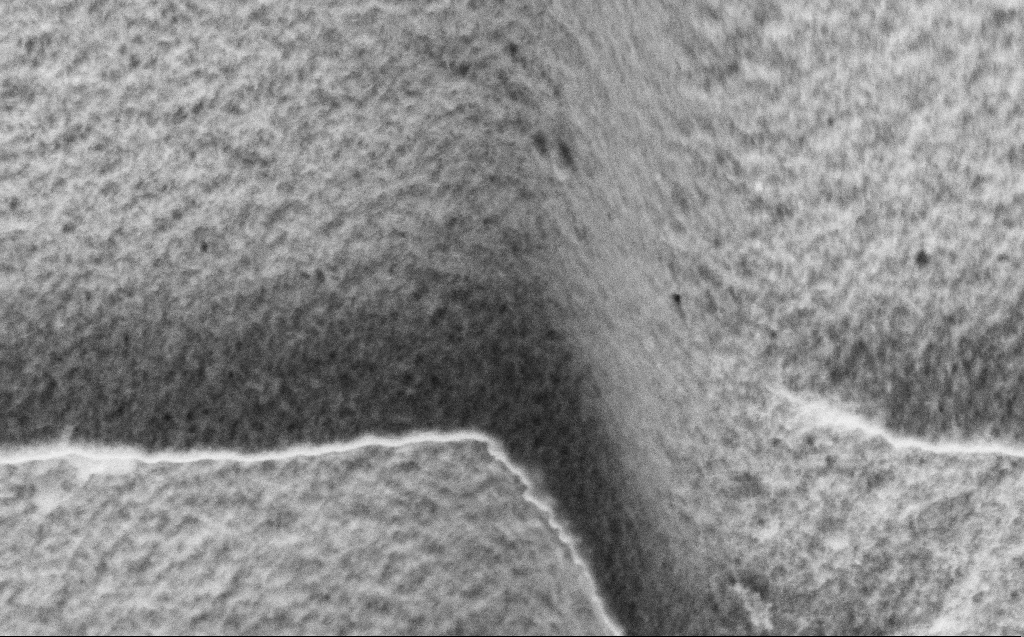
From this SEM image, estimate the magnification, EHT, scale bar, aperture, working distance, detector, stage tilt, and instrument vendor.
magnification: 61.43 K X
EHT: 3 kV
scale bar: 1000 nm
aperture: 30 µm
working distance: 4 mm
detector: SE2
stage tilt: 45°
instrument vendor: Zeiss